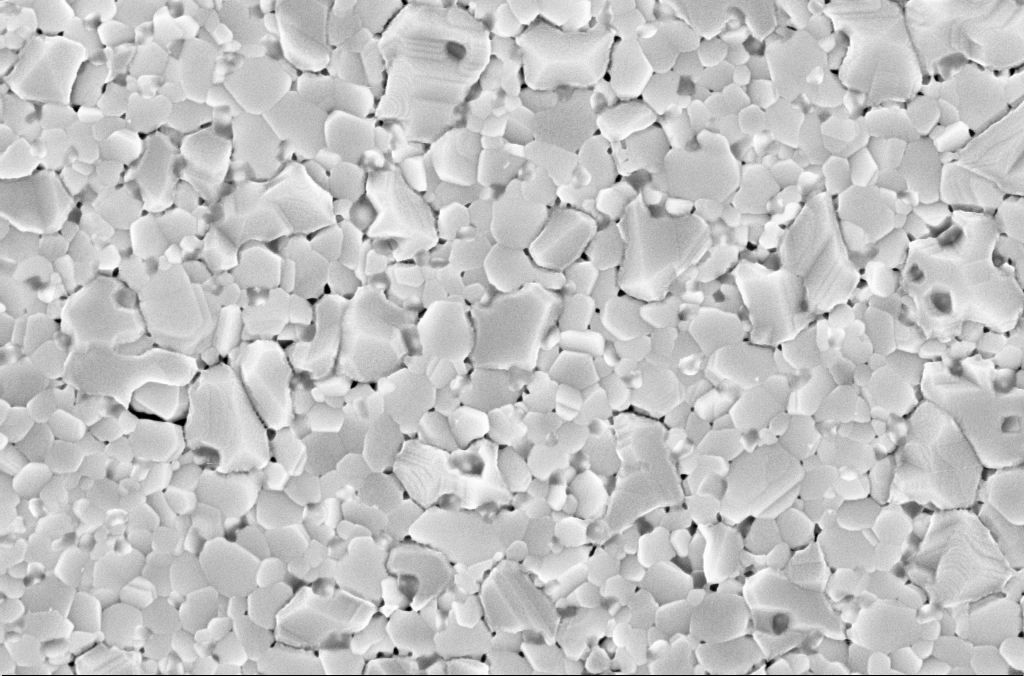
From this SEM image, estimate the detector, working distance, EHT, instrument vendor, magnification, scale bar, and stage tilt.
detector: InLens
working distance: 3 mm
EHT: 5 kV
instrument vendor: Zeiss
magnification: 70 K X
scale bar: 200 nm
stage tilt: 0°